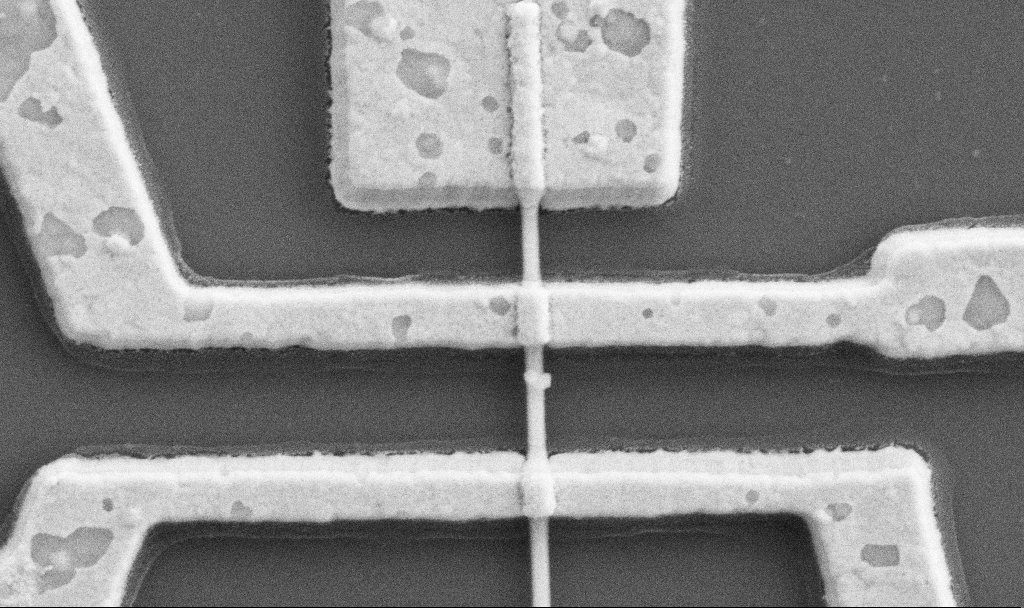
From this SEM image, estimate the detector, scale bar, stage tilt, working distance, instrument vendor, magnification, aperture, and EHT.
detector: SE2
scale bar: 1000 nm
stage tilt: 0°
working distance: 9.7 mm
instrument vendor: Zeiss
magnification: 60 K X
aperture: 30 µm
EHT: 5 kV